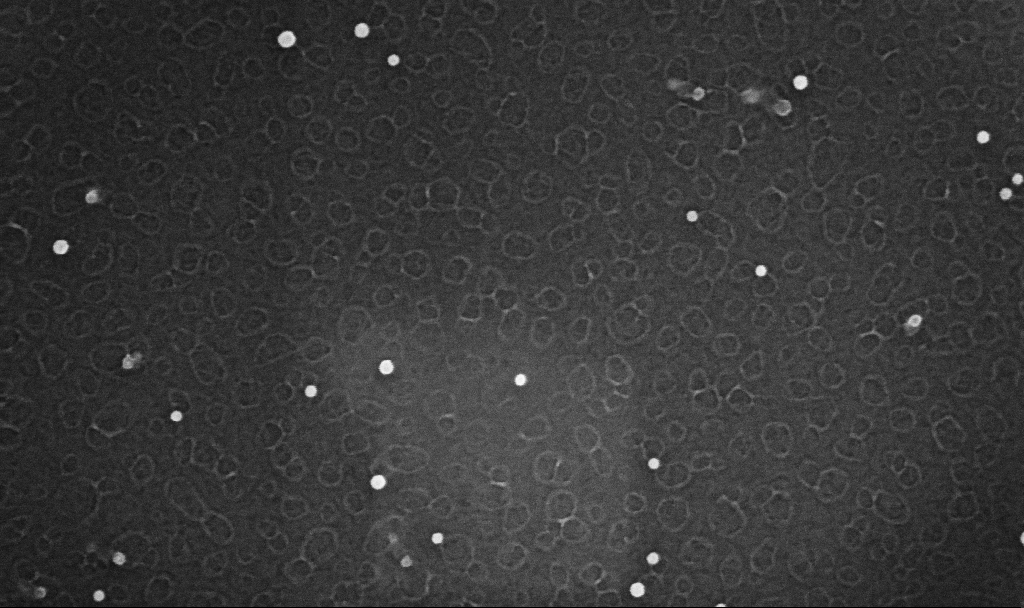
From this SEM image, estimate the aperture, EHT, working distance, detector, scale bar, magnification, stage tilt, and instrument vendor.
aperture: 30 µm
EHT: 10 kV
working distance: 3.3 mm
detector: InLens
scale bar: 100 nm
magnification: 200 K X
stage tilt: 0°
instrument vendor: Zeiss